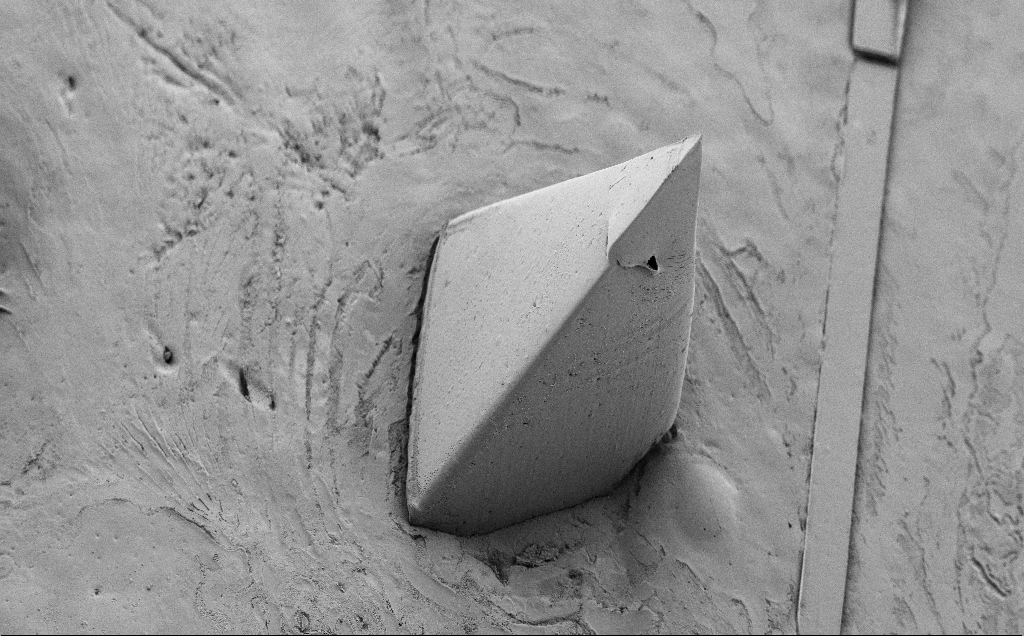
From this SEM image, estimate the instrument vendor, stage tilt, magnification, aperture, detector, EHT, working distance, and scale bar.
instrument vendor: Zeiss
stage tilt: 40°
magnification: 0.181 K X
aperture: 30 µm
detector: SE2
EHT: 5 kV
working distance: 8 mm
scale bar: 100000 nm